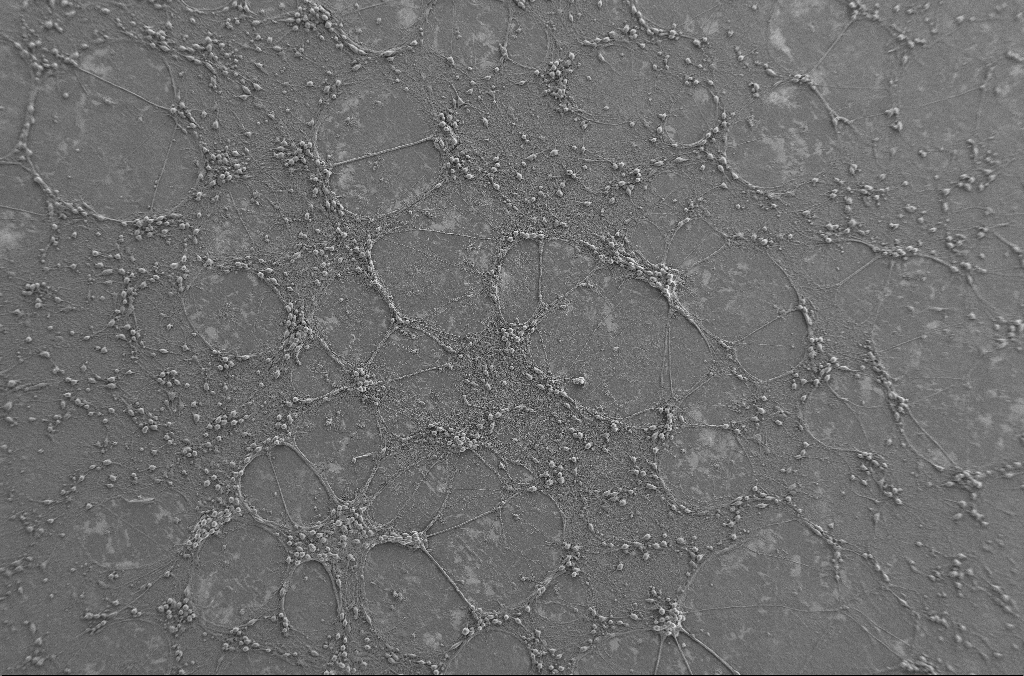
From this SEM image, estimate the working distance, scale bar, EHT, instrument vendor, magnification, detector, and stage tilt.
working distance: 4.1 mm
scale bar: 100000 nm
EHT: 2 kV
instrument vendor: Zeiss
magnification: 0.25 K X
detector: SE2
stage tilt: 0°